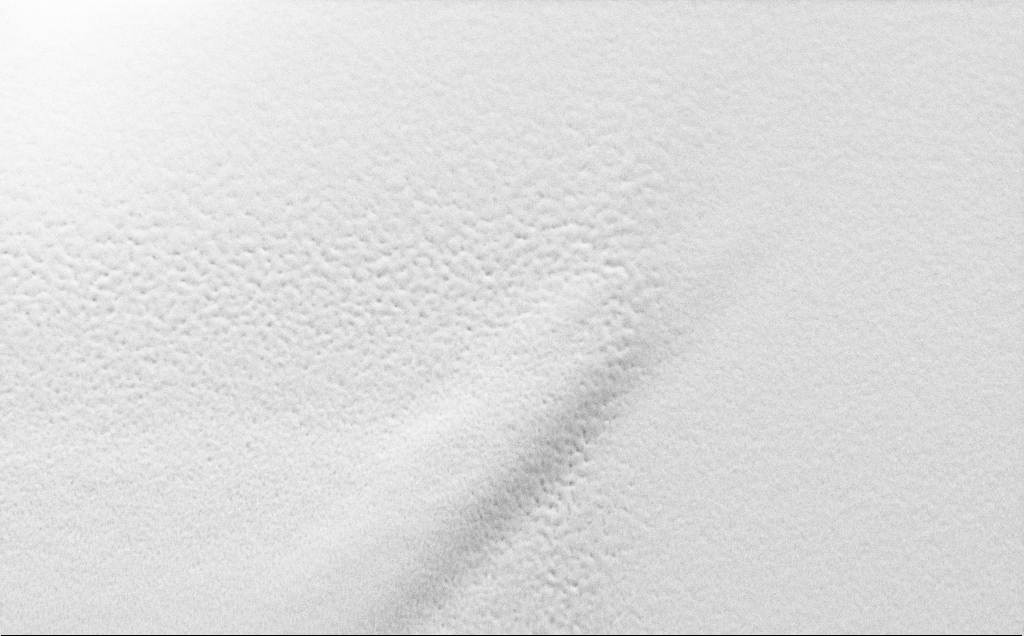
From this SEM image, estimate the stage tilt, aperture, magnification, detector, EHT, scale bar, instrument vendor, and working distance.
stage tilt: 45°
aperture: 30 µm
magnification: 24.01 K X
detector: SE2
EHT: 1.5 kV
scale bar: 2000 nm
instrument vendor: Zeiss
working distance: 5 mm